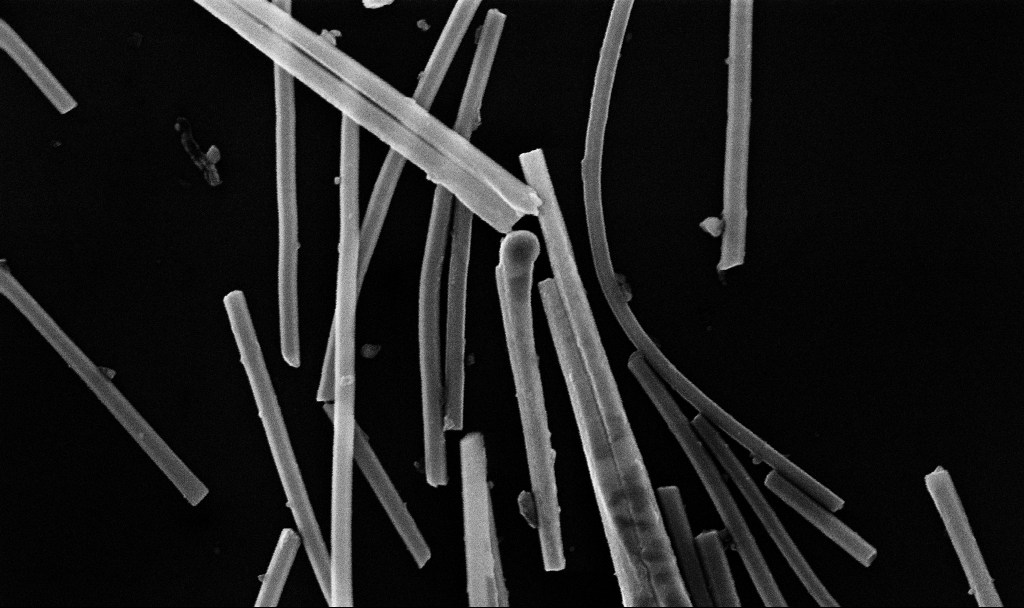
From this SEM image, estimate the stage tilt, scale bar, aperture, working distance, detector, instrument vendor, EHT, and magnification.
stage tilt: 0°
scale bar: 200 nm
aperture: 30 µm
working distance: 10.7 mm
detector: InLens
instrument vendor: Zeiss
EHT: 10 kV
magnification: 75.84 K X